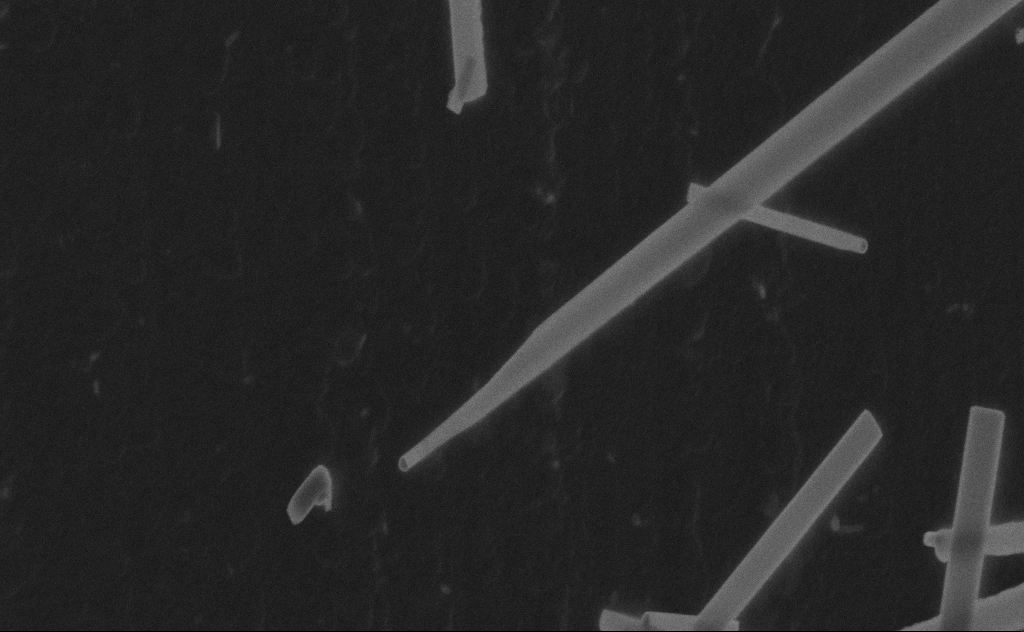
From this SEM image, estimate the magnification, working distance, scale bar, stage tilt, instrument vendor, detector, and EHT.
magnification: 108.06 K X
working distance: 9 mm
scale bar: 200 nm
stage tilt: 0°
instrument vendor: Zeiss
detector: SE2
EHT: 20 kV